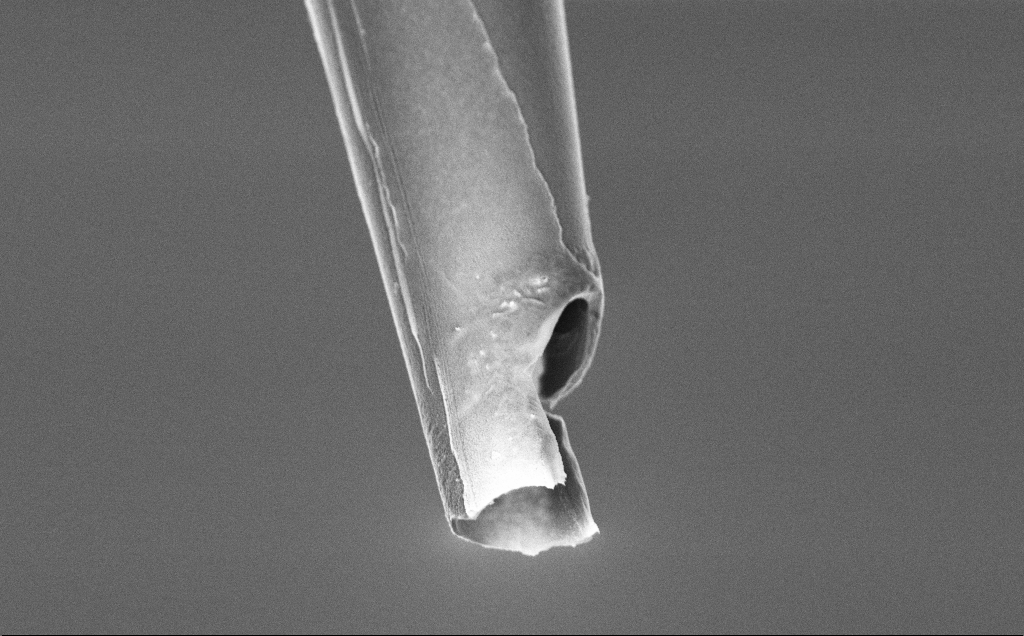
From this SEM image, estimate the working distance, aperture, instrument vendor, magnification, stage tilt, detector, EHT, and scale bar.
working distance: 7.7 mm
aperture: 30 µm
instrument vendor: Zeiss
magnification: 15 K X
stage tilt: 45°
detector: InLens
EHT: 3 kV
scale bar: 1000 nm